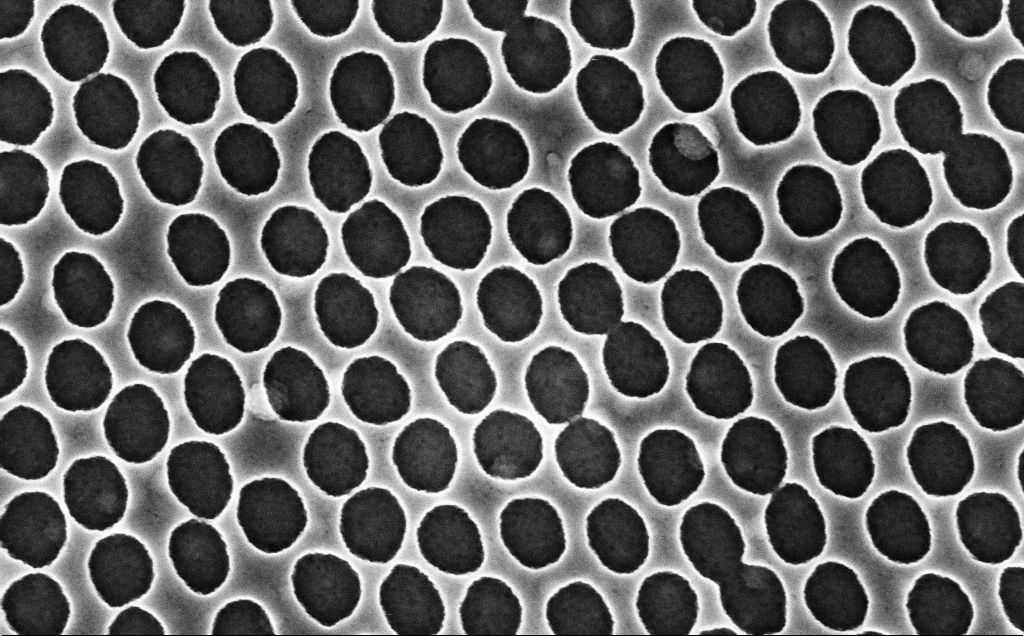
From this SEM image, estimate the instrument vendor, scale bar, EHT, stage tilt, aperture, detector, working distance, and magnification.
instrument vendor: Zeiss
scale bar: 200 nm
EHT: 3 kV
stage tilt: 0°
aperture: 30 µm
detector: InLens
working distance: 2.4 mm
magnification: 90 K X